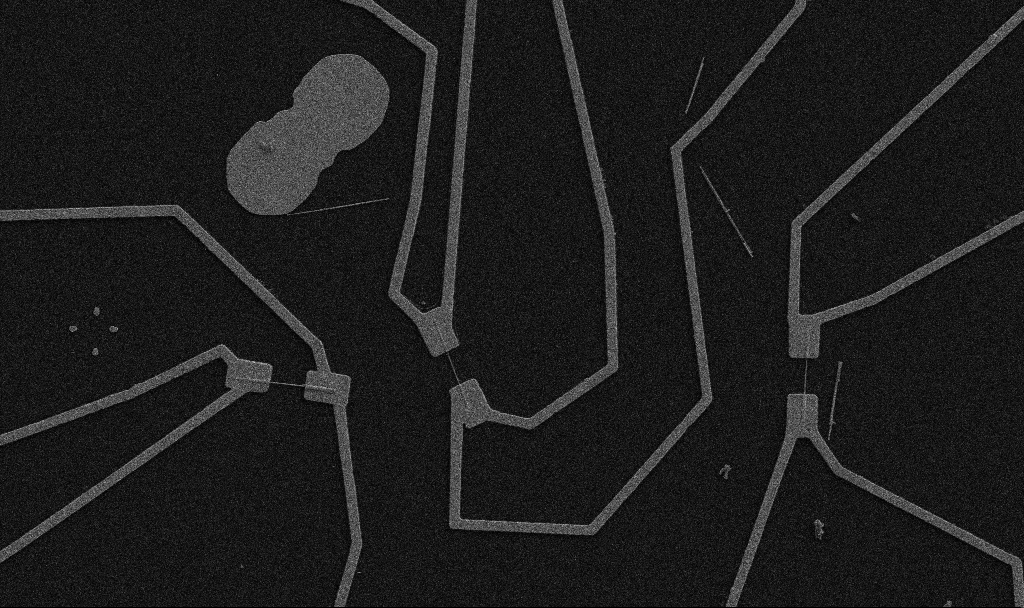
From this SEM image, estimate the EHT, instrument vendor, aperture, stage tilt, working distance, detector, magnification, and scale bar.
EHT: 5 kV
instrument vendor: Zeiss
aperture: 30 µm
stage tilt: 0°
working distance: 10.7 mm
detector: SE2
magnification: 5 K X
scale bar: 10000 nm